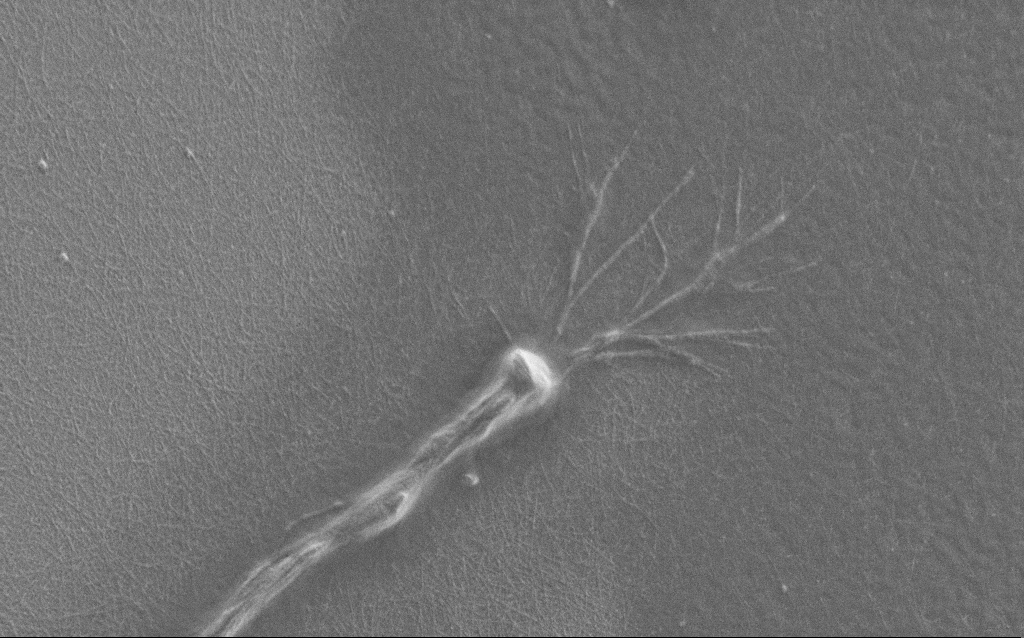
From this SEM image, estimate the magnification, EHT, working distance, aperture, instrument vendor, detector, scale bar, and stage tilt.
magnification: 7.5 K X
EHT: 1 kV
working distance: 6 mm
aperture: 30 µm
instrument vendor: Zeiss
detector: SE2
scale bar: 2000 nm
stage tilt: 0°